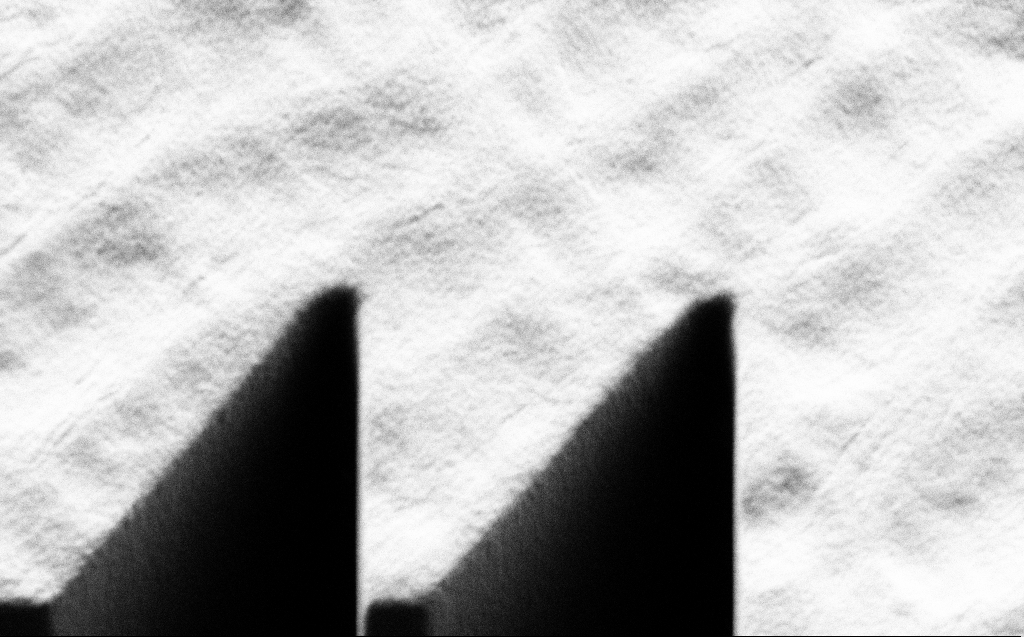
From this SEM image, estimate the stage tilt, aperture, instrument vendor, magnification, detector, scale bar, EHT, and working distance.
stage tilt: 45°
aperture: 30 µm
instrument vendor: Zeiss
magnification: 12.18 K X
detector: SE2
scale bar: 2000 nm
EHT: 1 kV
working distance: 6 mm